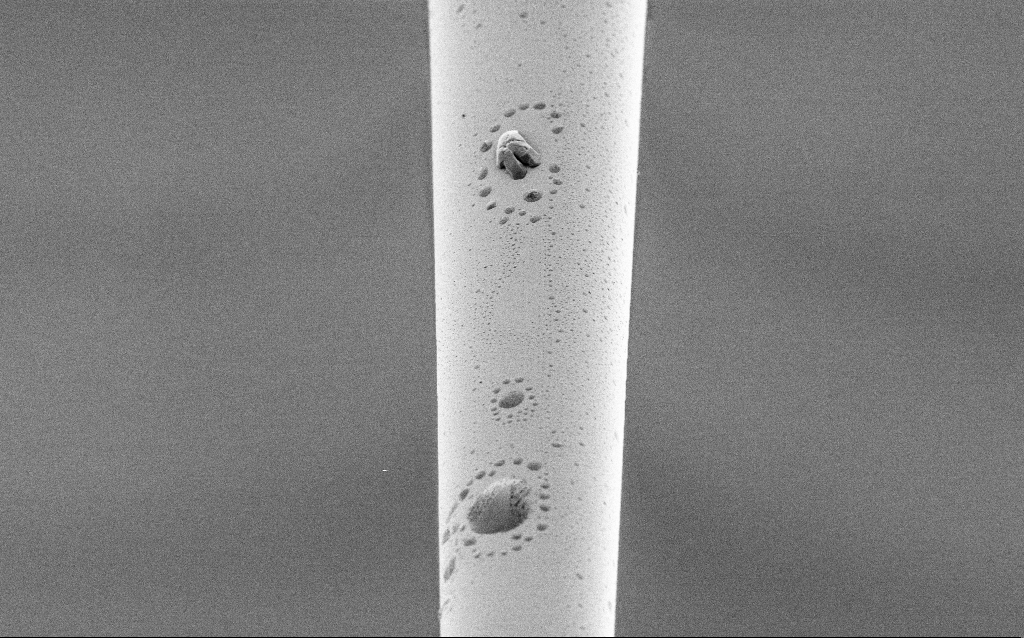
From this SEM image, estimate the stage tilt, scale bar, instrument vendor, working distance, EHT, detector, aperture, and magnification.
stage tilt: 45°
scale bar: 10000 nm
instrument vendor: Zeiss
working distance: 6.5 mm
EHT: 1 kV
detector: SE2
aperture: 30 µm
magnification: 1.5 K X